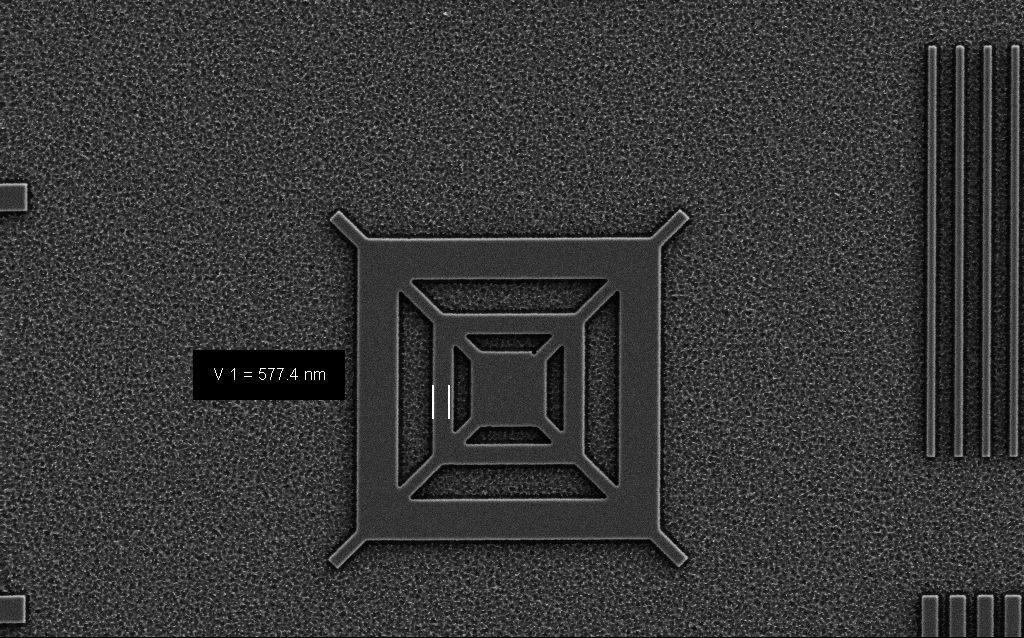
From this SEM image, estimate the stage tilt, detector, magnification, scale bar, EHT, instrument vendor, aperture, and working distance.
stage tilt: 0°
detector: SE2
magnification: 10.17 K X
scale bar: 2000 nm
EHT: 3 kV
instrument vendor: Zeiss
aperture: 30 µm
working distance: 5 mm